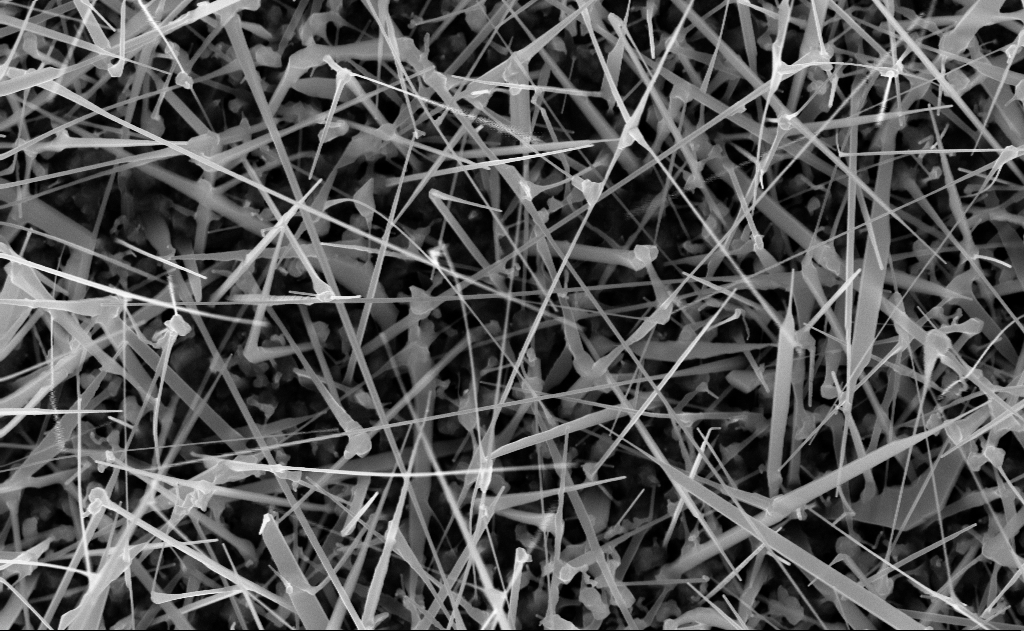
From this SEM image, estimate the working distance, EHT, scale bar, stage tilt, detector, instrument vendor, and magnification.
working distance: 10 mm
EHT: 10 kV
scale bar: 1000 nm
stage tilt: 0°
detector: InLens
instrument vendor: Zeiss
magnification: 40 K X